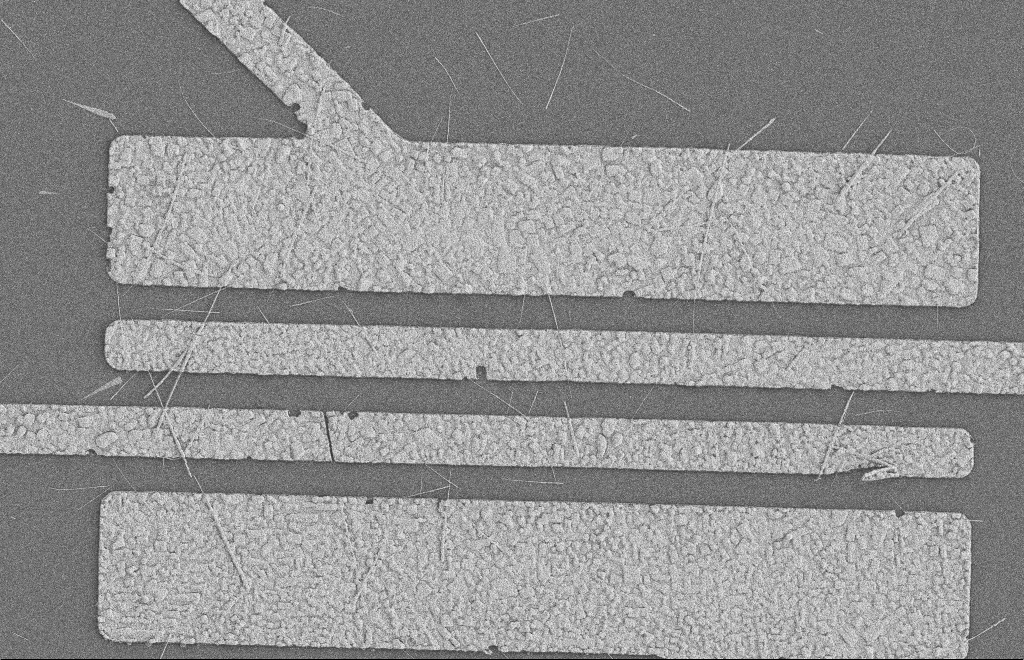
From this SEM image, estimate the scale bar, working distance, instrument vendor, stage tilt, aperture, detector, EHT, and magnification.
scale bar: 2000 nm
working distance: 8 mm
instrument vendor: Zeiss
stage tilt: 0°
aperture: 20 µm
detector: SE2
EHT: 2 kV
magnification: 5.24 K X